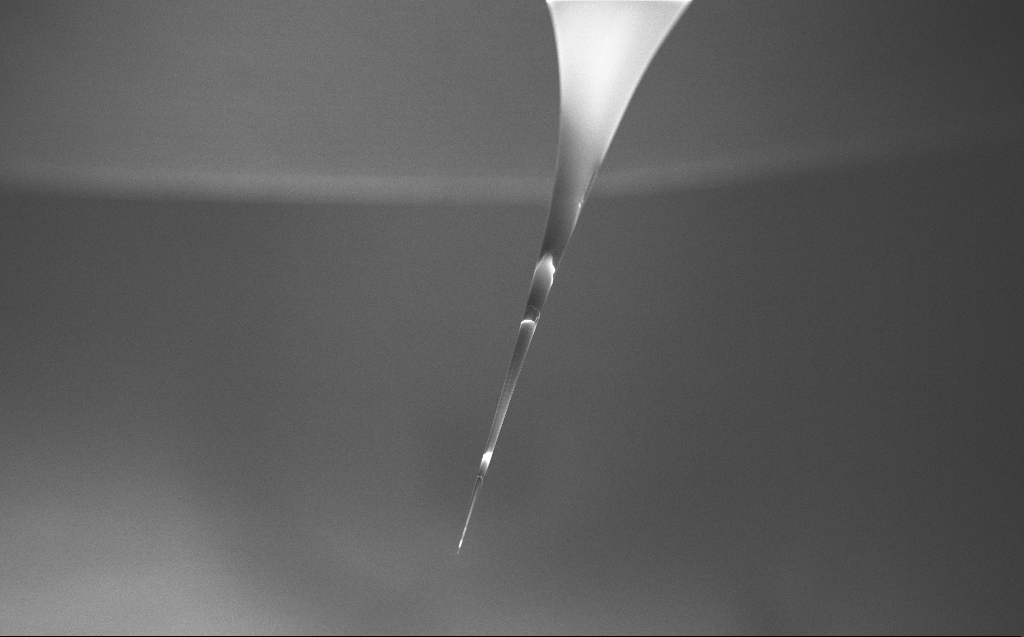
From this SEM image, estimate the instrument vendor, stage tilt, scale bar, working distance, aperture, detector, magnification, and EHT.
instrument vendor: Zeiss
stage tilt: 45°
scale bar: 200000 nm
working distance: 6 mm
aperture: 30 µm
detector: InLens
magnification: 0.1 K X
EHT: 5 kV